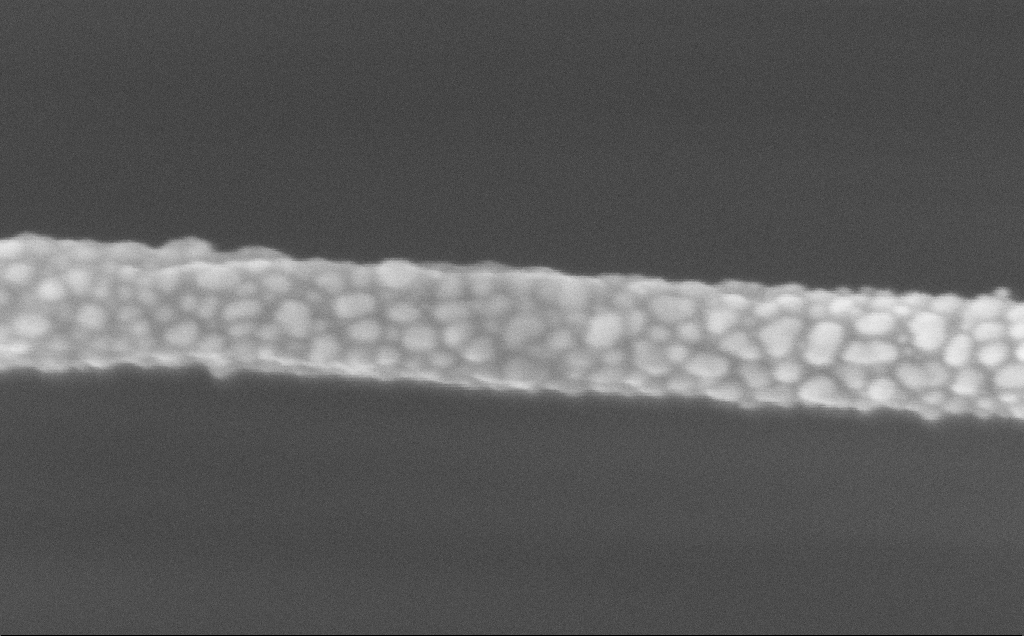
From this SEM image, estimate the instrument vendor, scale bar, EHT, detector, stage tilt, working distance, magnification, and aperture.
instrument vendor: Zeiss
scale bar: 100 nm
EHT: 5 kV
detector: InLens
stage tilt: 0°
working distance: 12 mm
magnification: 348.46 K X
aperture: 30 µm